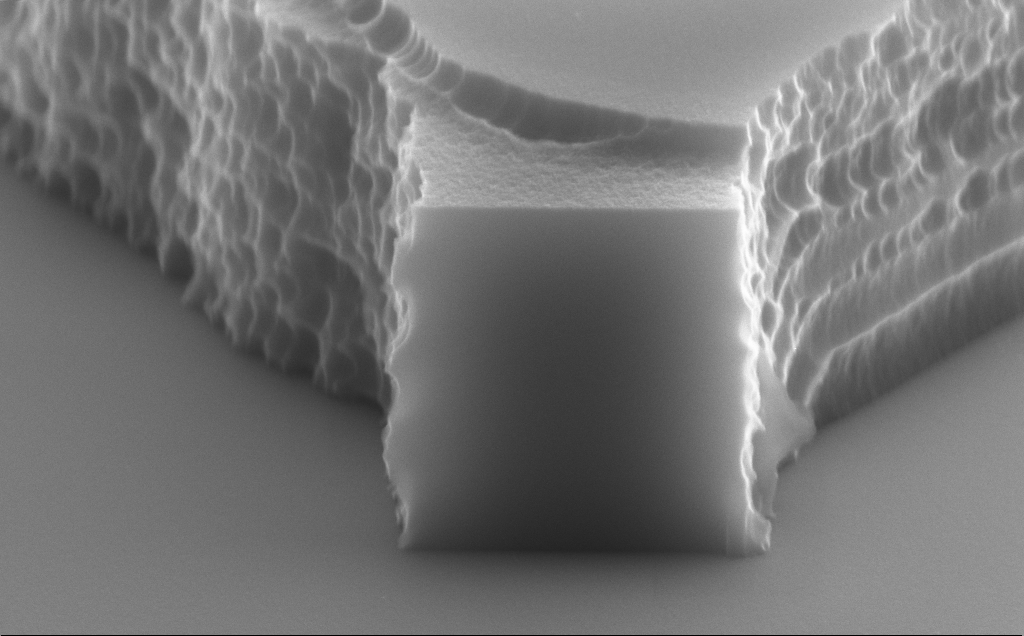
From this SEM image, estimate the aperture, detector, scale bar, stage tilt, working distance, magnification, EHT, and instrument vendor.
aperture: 30 µm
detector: SE2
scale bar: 1000 nm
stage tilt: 70°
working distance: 10 mm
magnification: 59.96 K X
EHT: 10 kV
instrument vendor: Zeiss